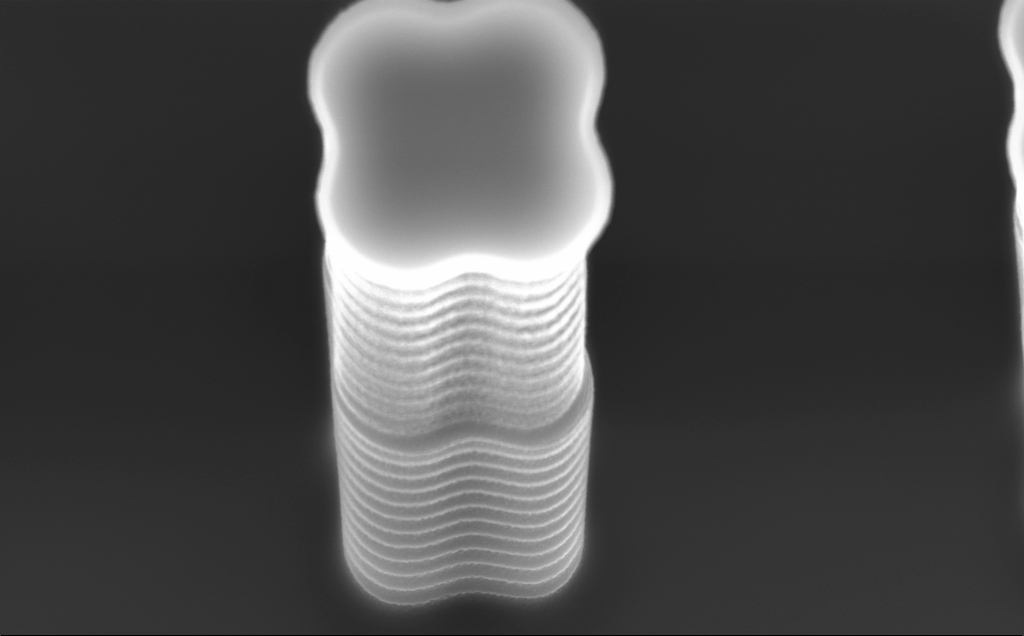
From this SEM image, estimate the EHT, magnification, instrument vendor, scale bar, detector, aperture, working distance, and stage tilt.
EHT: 10 kV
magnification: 25.39 K X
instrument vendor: Zeiss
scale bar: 2000 nm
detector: InLens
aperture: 120 µm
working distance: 9 mm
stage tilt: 30.3°